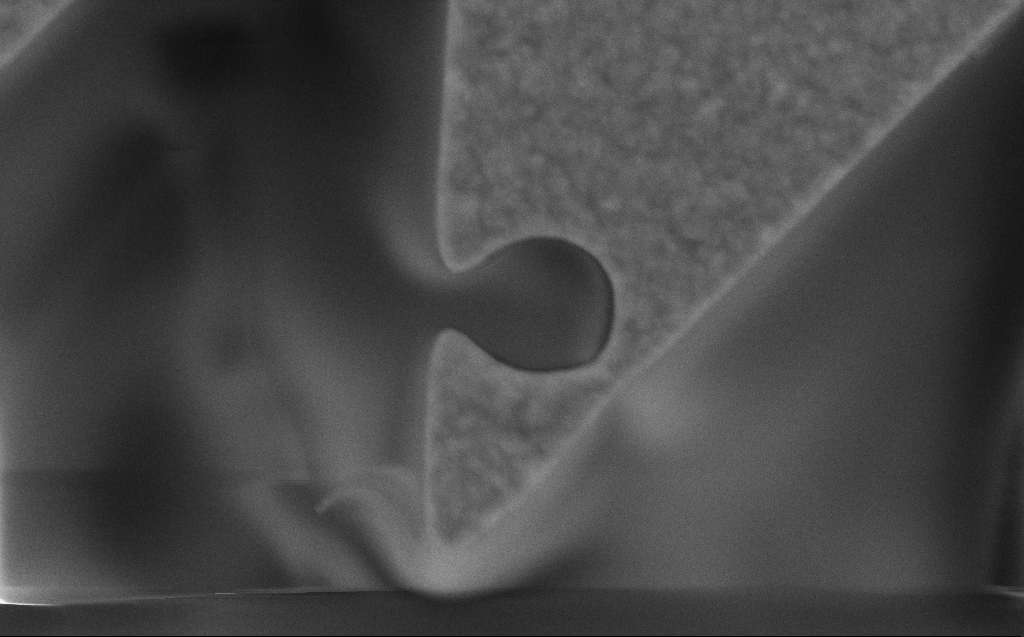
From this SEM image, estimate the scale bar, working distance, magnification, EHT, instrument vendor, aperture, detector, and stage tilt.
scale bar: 2000 nm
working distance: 8 mm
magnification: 28.61 K X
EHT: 10 kV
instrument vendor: Zeiss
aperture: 30 µm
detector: SE2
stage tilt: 0°